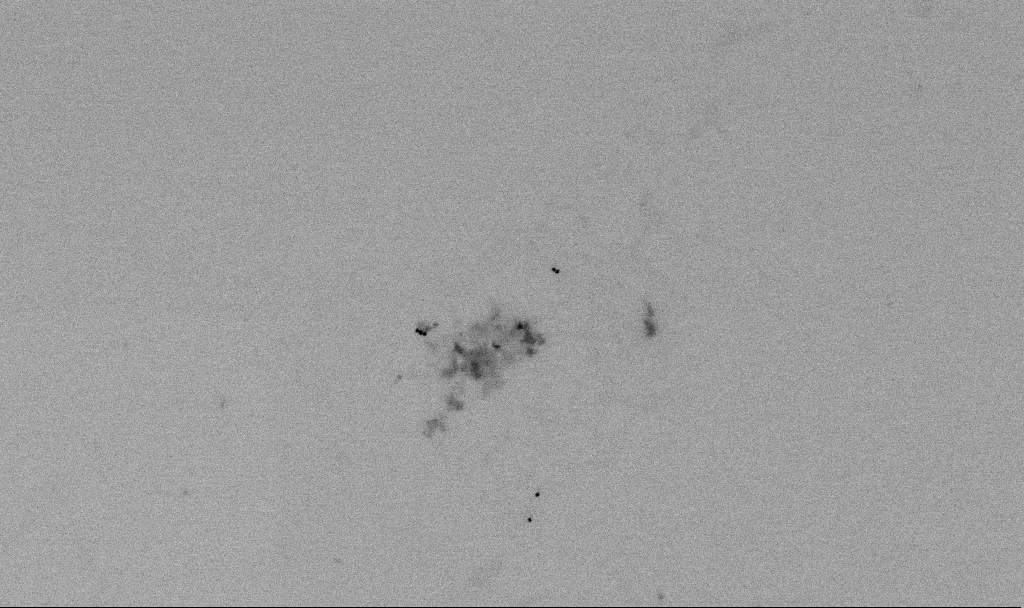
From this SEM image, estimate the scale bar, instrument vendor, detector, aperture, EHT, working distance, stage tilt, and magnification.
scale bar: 1000 nm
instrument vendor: Zeiss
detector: SE2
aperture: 30 µm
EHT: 2 kV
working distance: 5.5 mm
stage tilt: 0°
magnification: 60 K X